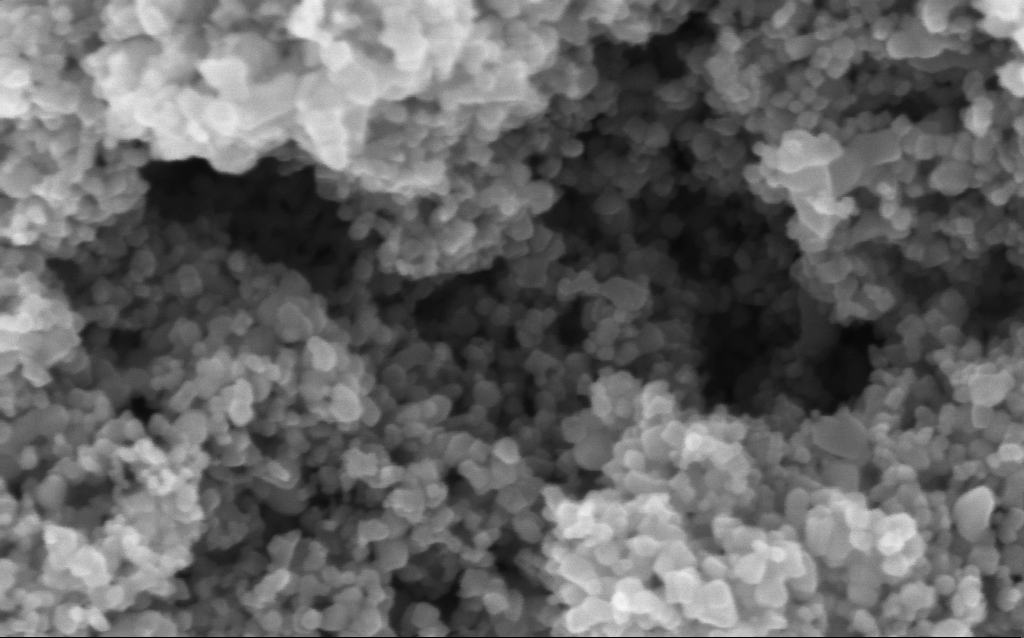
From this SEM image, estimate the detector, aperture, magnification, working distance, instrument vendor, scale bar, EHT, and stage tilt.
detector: InLens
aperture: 30 µm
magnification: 294.6 K X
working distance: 4.5 mm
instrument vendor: Zeiss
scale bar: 200 nm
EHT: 5 kV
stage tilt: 0°